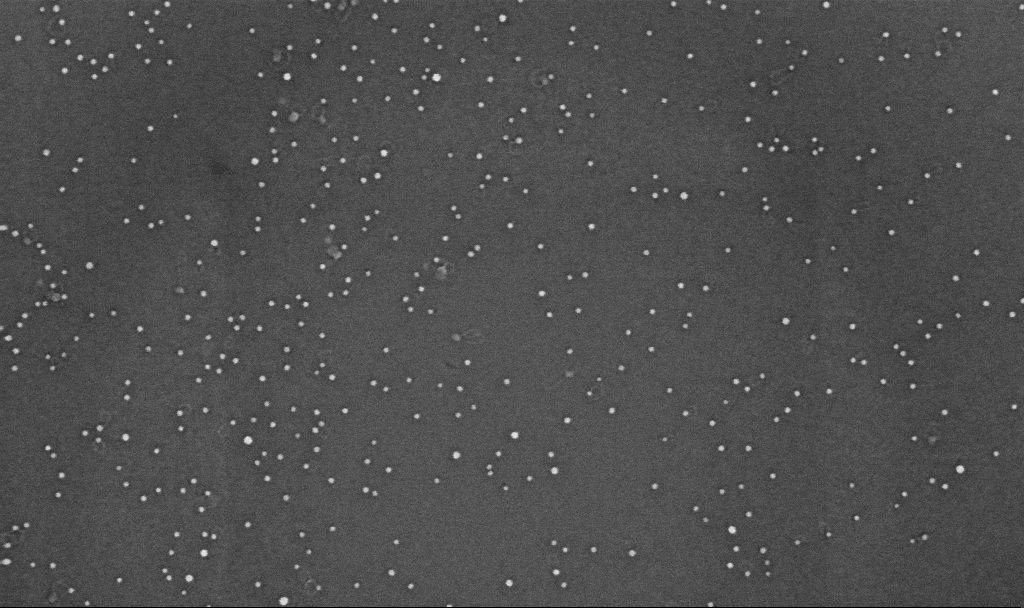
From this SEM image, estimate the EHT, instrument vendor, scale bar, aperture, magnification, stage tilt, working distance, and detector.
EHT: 10 kV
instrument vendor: Zeiss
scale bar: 100 nm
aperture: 30 µm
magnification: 200 K X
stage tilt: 0°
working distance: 3.3 mm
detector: InLens